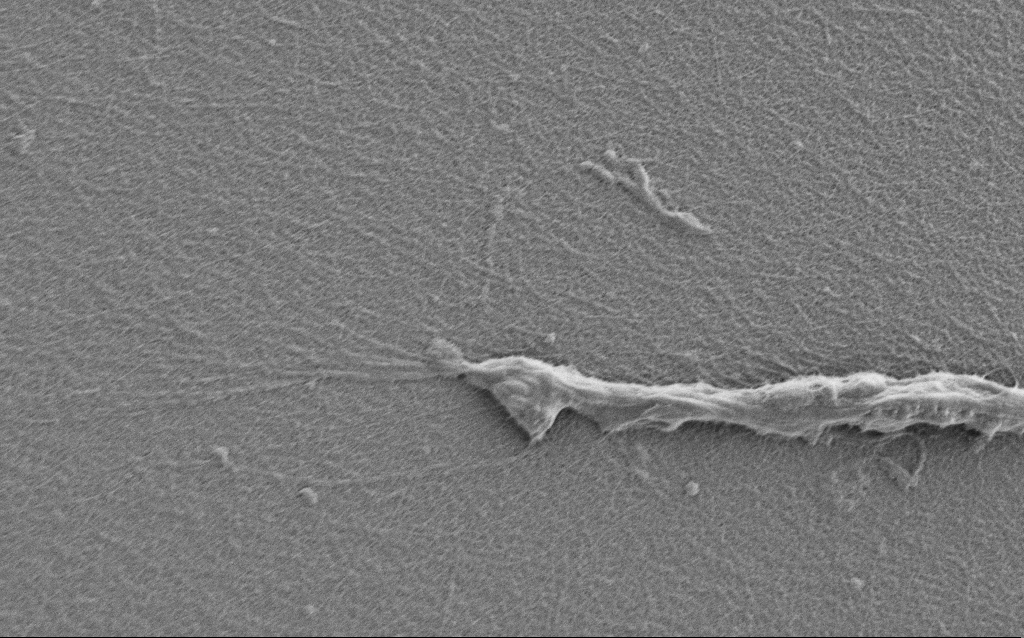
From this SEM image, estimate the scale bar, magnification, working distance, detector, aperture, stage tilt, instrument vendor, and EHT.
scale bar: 2000 nm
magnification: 10 K X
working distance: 7 mm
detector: SE2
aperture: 30 µm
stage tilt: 0°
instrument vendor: Zeiss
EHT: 0.9 kV